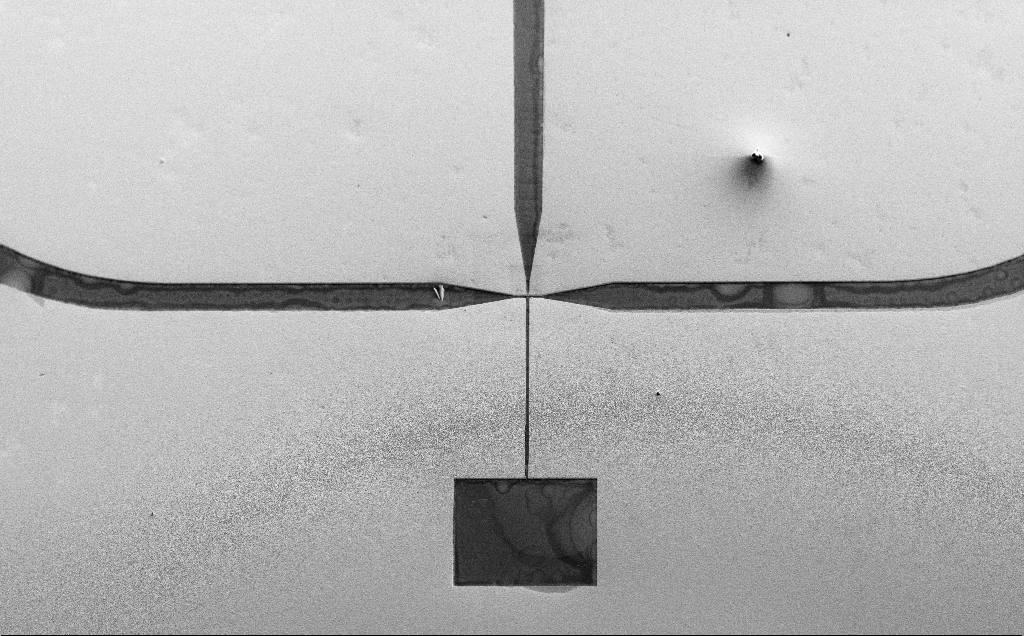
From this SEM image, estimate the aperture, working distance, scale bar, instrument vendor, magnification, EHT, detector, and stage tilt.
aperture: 30 µm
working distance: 15 mm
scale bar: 100000 nm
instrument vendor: Zeiss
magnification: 0.134 K X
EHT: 10 kV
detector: SE2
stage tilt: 45°